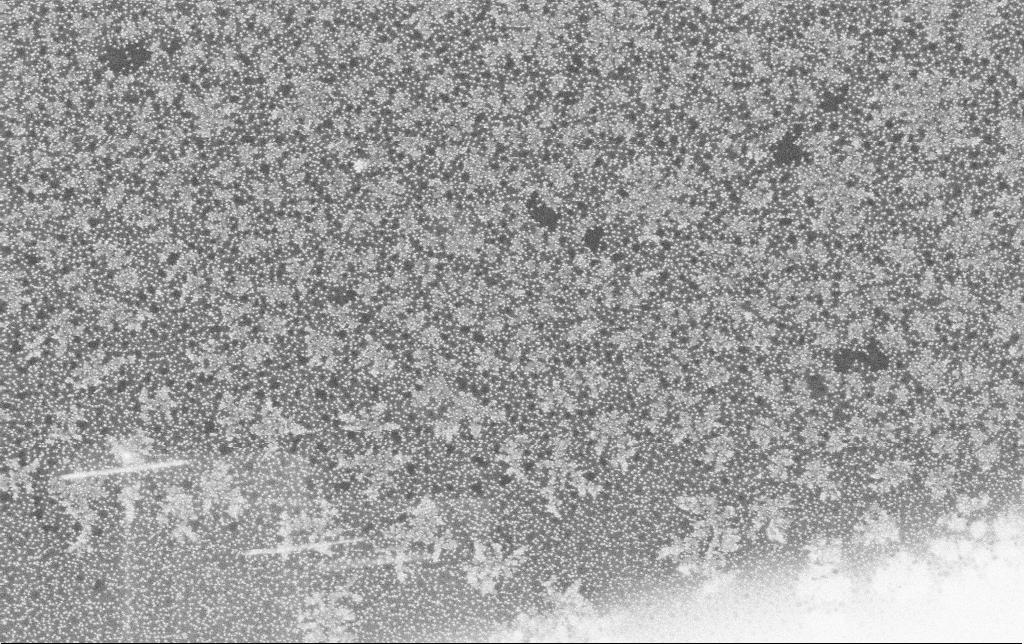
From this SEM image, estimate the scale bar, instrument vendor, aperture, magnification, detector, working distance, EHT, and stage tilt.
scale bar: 1000 nm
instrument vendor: Zeiss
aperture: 30 µm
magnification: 51.05 K X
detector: SE2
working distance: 11.3 mm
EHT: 8 kV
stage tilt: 0°